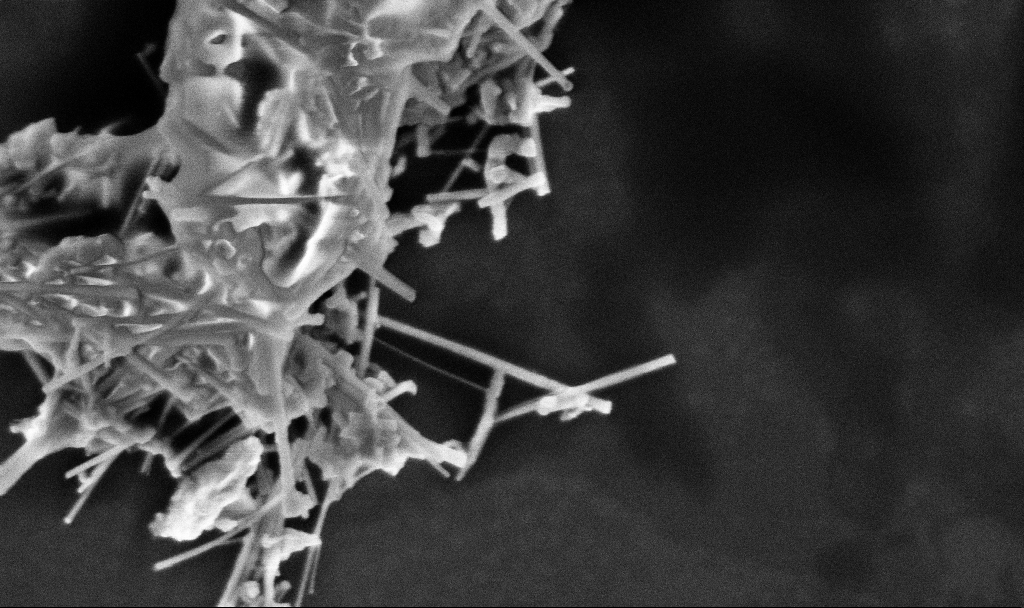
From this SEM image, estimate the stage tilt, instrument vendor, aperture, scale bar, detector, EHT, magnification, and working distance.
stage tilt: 0°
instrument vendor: Zeiss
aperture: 30 µm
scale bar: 200 nm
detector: InLens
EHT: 3 kV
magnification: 79.74 K X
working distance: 3.3 mm